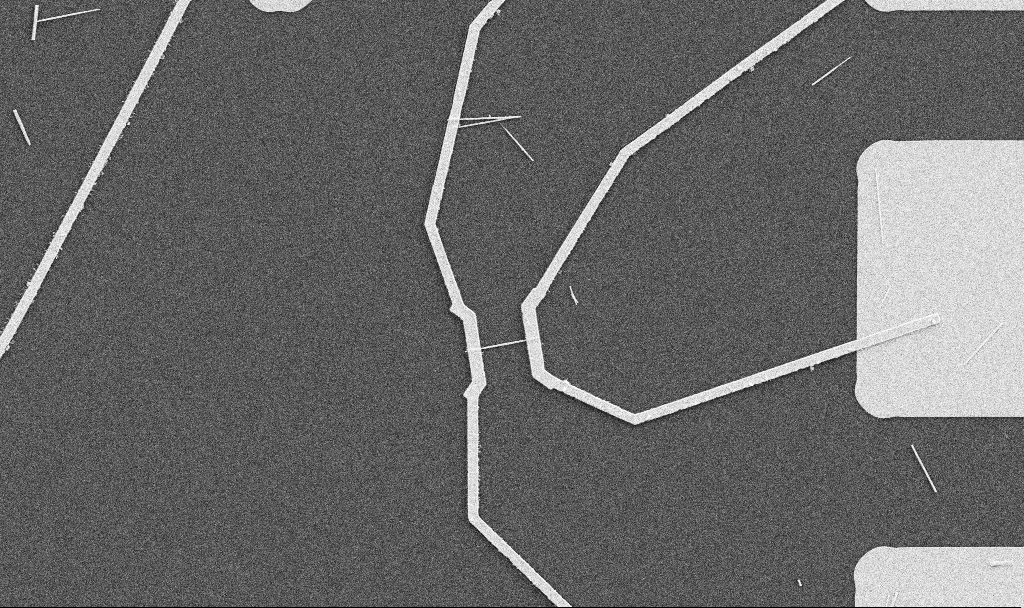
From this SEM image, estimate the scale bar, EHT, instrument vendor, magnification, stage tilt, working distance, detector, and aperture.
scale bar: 10000 nm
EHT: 5 kV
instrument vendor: Zeiss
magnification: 5 K X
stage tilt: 0°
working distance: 10.7 mm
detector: SE2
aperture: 30 µm